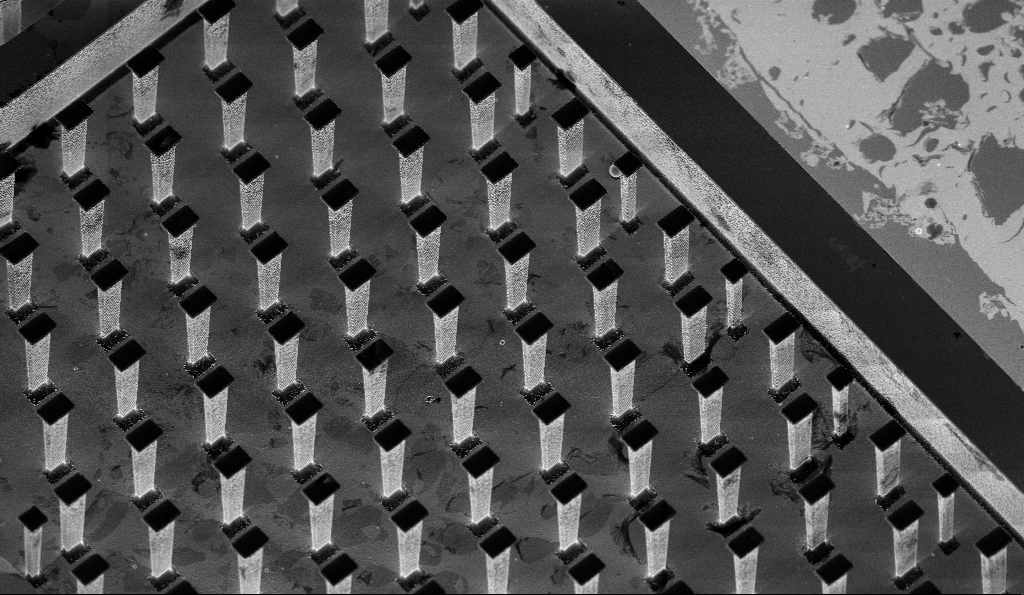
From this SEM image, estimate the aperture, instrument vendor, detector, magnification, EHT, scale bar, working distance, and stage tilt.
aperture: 30 µm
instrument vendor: Zeiss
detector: InLens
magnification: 2.84 K X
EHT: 3 kV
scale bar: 20000 nm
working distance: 5.5 mm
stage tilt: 30°